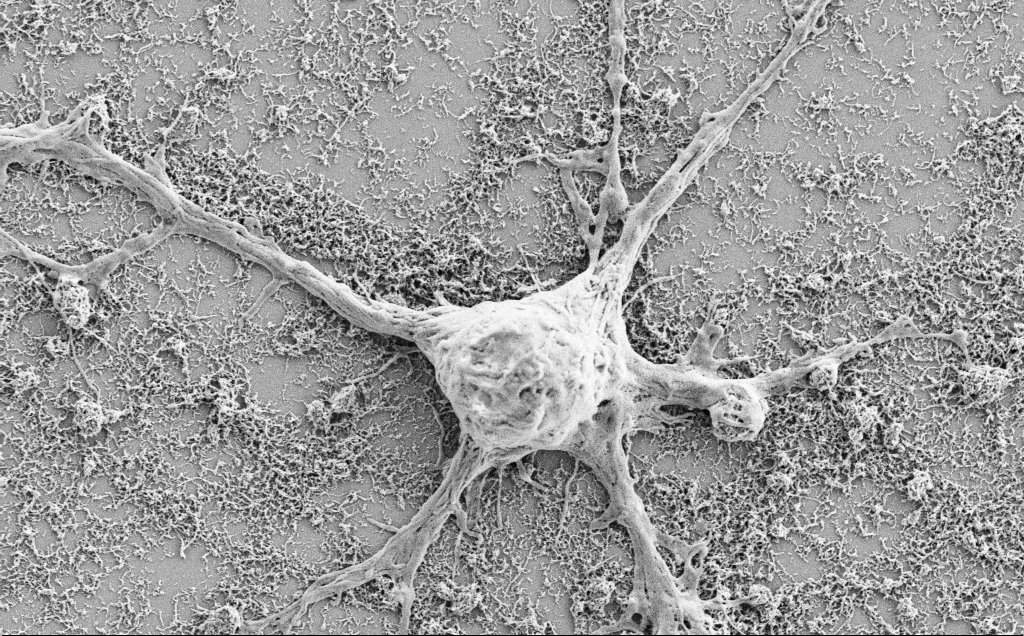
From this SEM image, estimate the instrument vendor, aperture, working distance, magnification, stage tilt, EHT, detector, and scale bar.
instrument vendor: Zeiss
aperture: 30 µm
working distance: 7.1 mm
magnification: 10 K X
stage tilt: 0°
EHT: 2 kV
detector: SE2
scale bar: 2000 nm